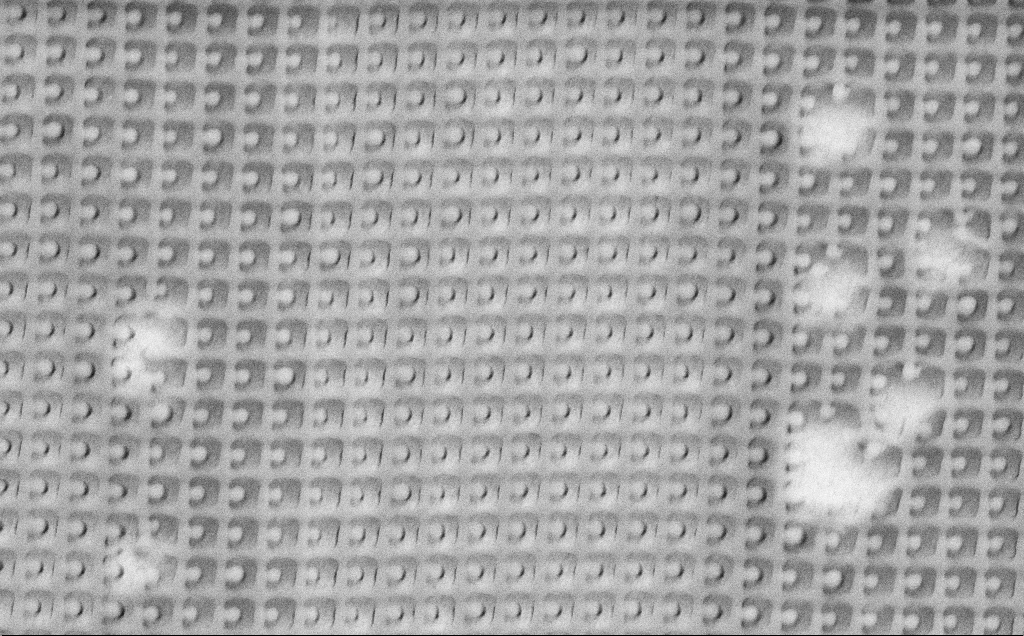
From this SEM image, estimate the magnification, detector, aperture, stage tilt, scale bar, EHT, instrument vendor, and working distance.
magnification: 31.43 K X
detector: SE2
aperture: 30 µm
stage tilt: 0°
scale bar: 1000 nm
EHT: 3 kV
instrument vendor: Zeiss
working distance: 8.1 mm